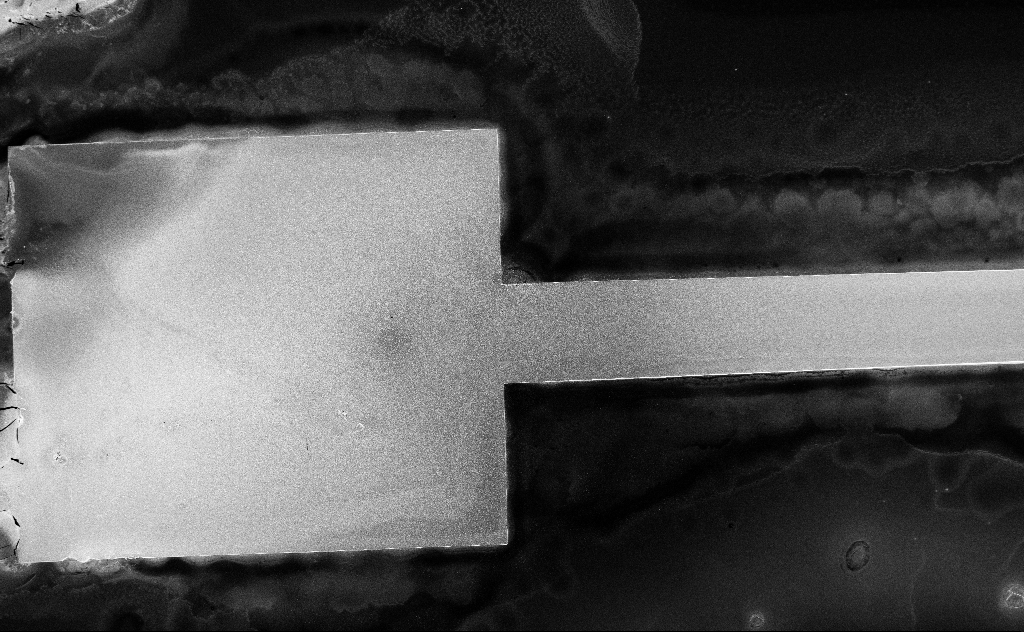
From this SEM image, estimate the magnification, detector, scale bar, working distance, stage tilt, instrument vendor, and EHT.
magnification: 0.275 K X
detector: InLens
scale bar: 100000 nm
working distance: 6 mm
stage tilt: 0°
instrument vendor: Zeiss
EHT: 5 kV